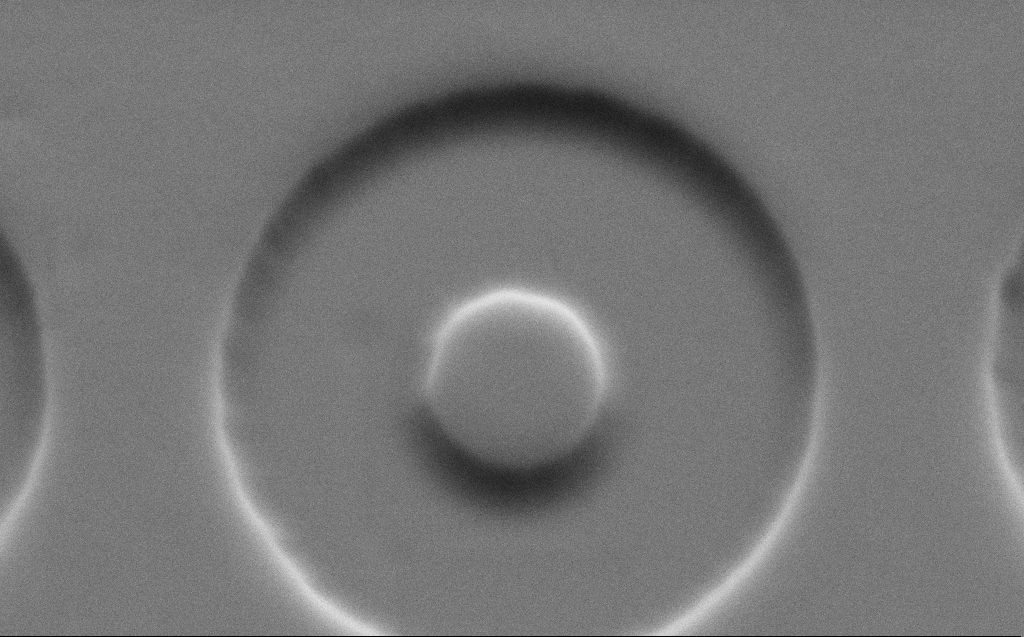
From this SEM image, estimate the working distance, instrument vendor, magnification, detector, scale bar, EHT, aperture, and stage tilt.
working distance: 6 mm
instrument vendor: Zeiss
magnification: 28.42 K X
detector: SE2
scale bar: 2000 nm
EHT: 5 kV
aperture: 30 µm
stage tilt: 45°